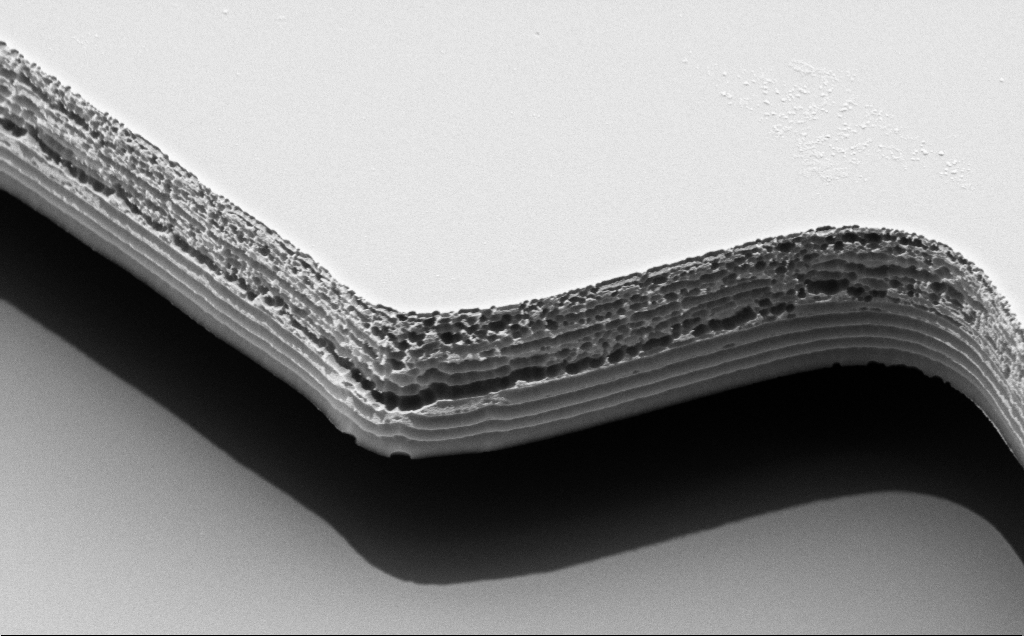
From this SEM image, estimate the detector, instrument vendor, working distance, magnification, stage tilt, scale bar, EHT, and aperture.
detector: SE2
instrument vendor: Zeiss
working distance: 10 mm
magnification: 27.36 K X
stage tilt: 50°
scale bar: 2000 nm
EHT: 5 kV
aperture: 30 µm